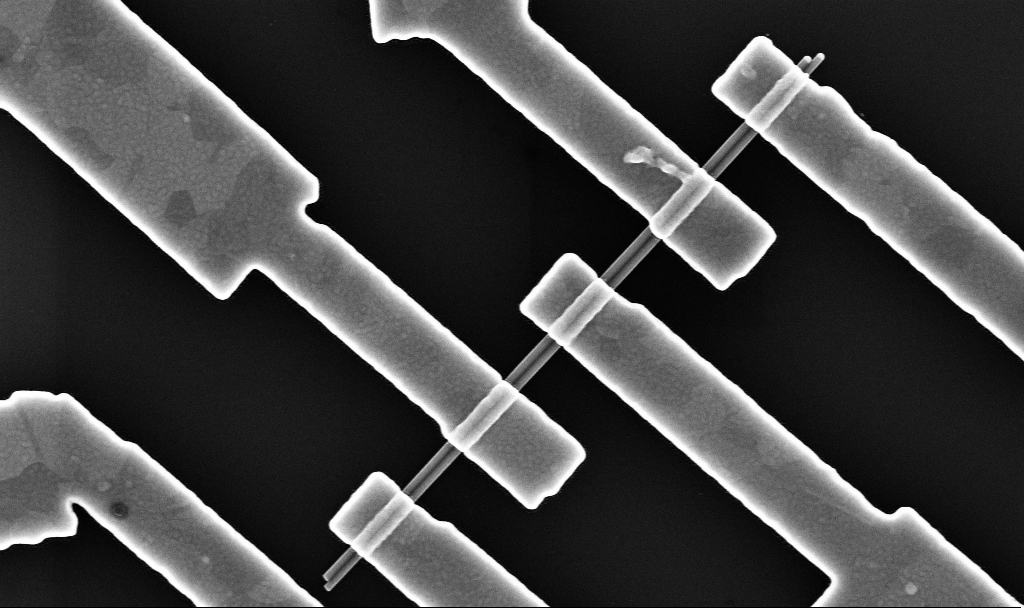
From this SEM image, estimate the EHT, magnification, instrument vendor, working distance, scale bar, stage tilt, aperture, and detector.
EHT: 10 kV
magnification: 53.3 K X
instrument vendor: Zeiss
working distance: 6.7 mm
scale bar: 1000 nm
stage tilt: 0°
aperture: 30 µm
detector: InLens